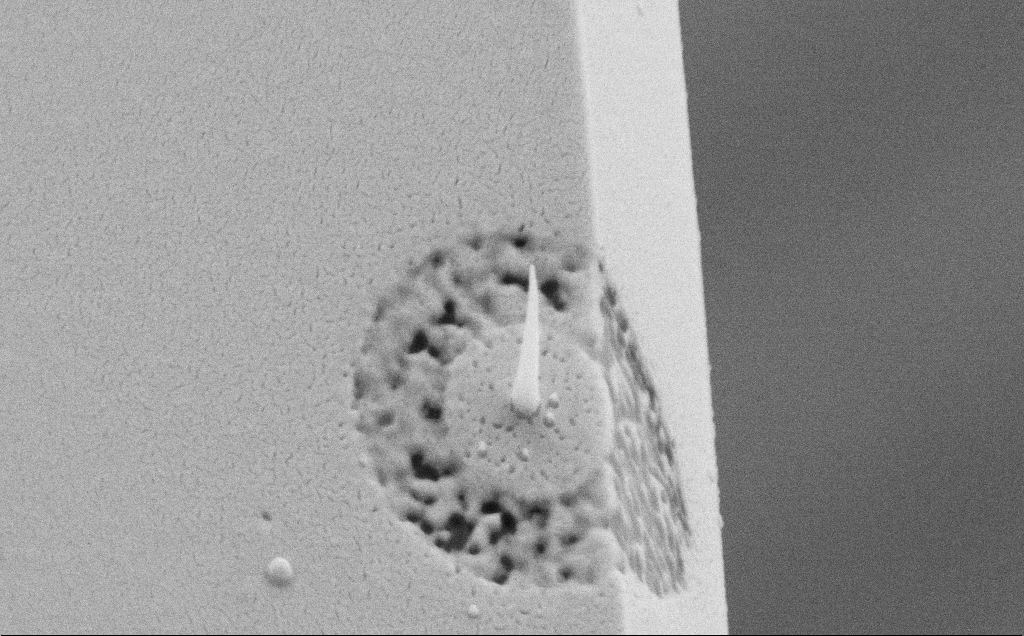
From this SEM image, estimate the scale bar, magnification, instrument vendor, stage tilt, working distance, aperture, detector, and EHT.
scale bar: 2000 nm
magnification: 32.22 K X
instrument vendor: Zeiss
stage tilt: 44.2°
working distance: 3 mm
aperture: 30 µm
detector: SE2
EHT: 10 kV